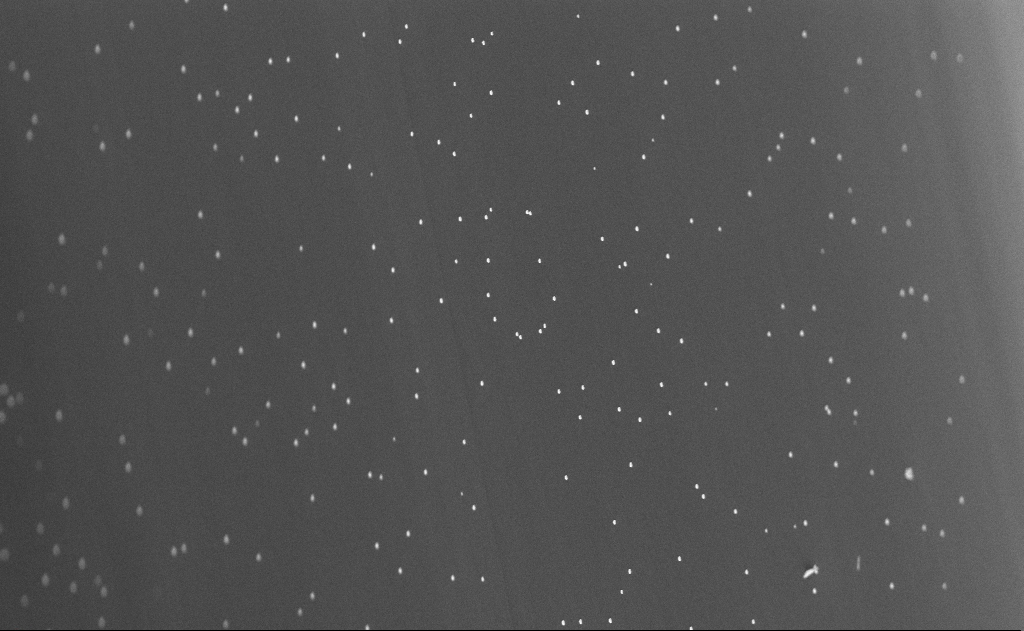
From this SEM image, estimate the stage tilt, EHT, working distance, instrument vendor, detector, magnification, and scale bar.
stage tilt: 0°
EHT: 10 kV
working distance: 10 mm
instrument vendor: Zeiss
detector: InLens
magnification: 20 K X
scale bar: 2000 nm